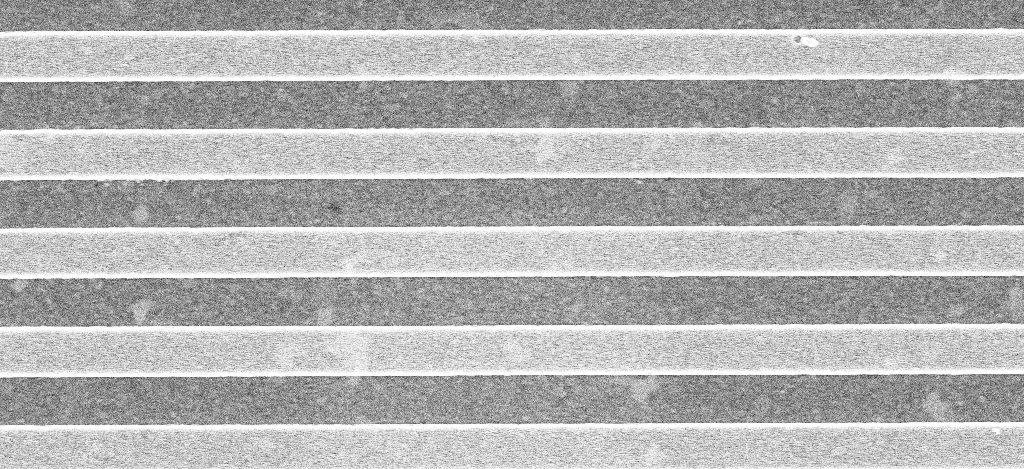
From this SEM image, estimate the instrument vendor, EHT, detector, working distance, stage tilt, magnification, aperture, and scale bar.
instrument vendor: Zeiss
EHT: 5 kV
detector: InLens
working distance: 3.1 mm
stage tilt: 0°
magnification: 40.48 K X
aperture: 30 µm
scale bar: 1000 nm